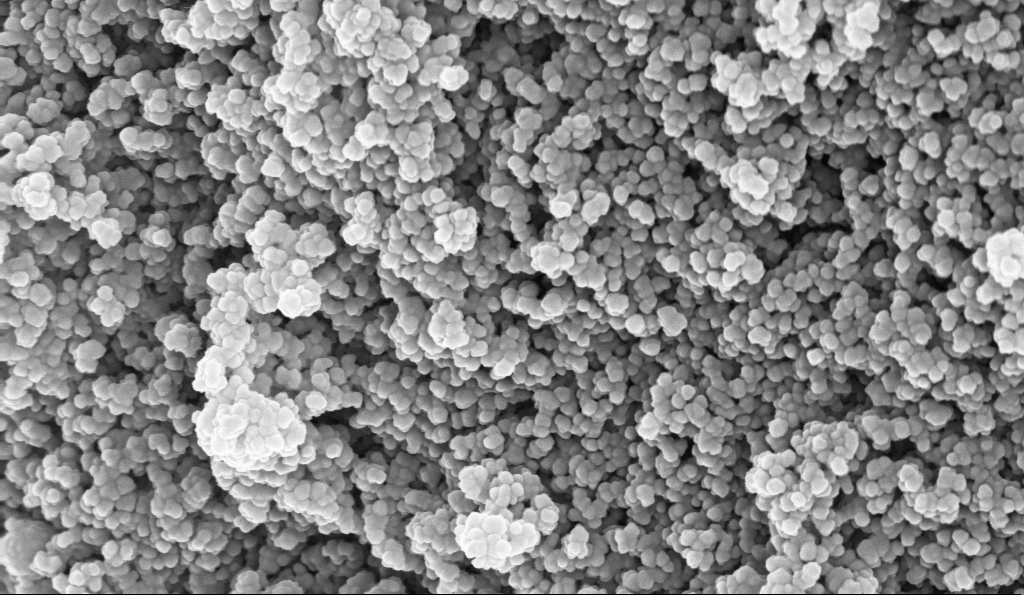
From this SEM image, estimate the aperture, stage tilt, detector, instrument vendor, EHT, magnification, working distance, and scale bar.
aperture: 30 µm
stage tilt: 0°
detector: InLens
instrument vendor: Zeiss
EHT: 10 kV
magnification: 135 K X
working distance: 5.1 mm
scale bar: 100 nm